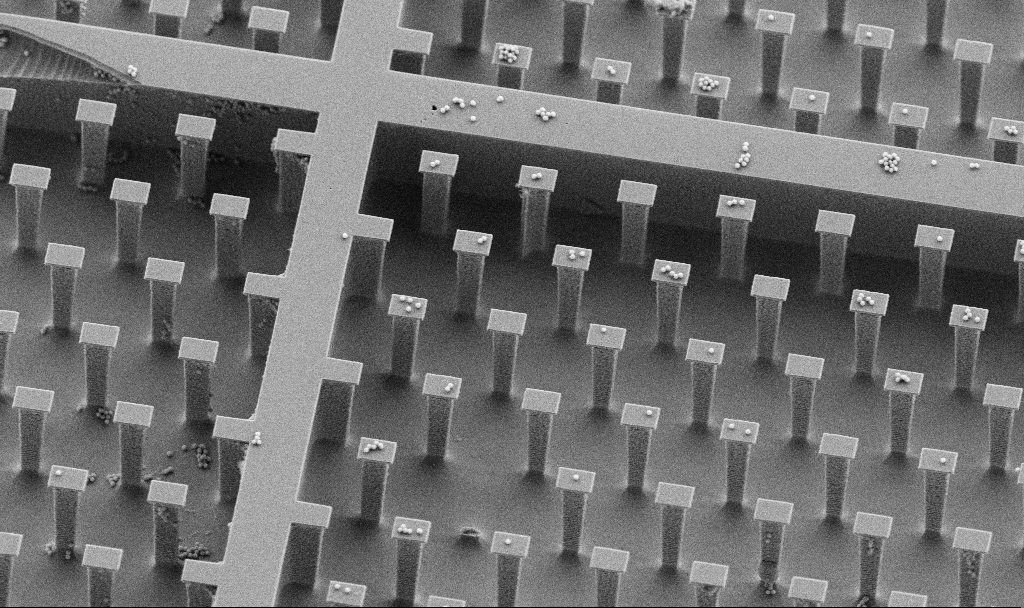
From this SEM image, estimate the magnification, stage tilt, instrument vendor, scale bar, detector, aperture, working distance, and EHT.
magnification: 3.08 K X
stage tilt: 30°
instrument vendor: Zeiss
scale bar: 10000 nm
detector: SE2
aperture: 30 µm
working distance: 4.5 mm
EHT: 5 kV